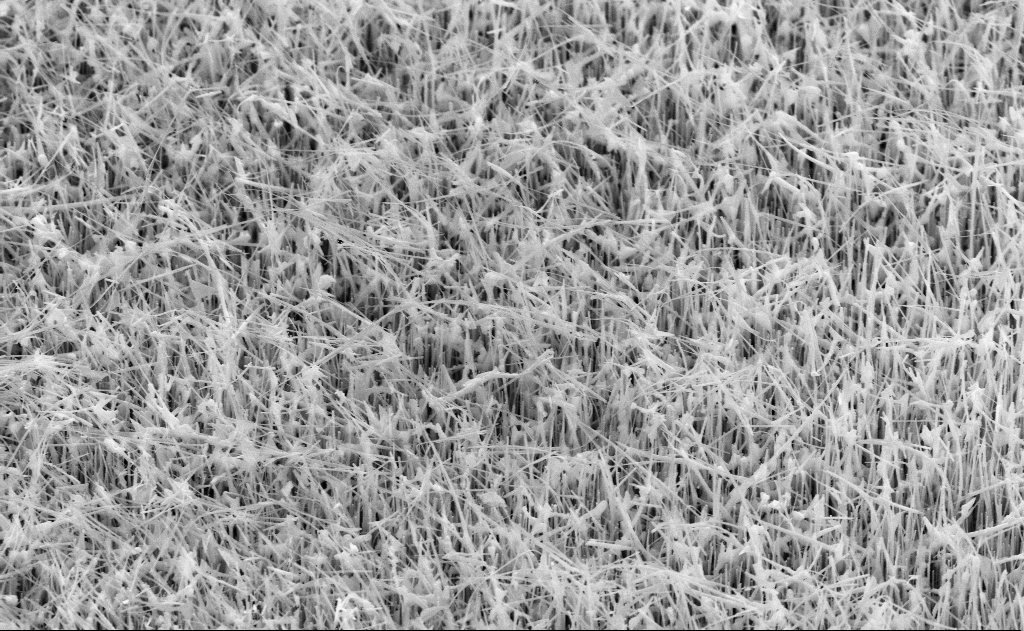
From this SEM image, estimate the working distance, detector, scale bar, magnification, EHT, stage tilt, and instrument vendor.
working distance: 14 mm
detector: SE2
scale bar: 2000 nm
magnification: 20 K X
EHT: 10 kV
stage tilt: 45°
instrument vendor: Zeiss